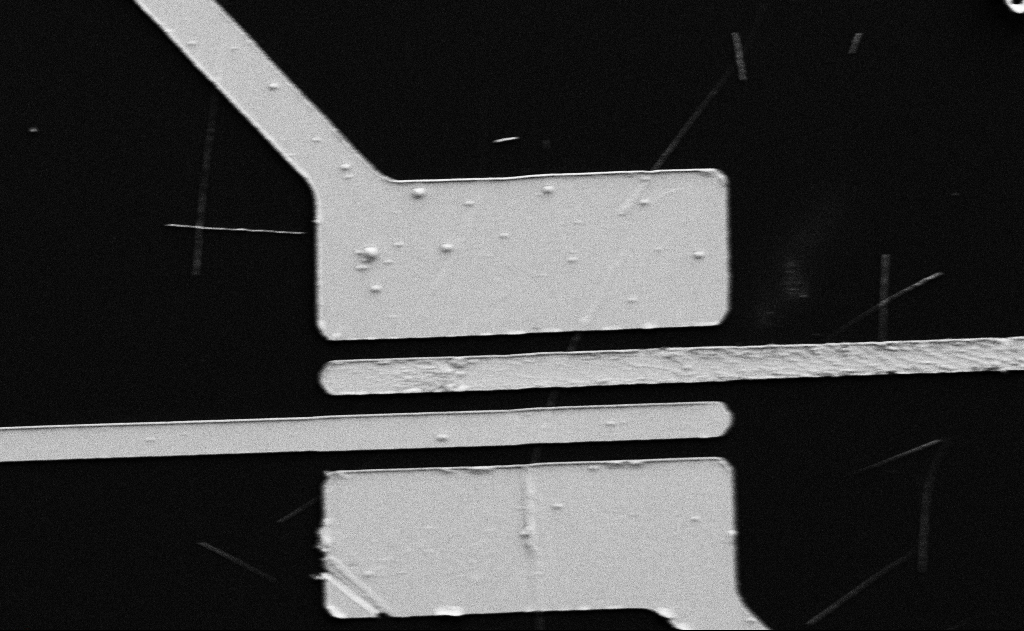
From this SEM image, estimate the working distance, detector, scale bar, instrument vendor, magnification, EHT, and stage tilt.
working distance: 15 mm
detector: SE2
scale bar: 10000 nm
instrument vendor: Zeiss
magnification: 5 K X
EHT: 5 kV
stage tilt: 0°